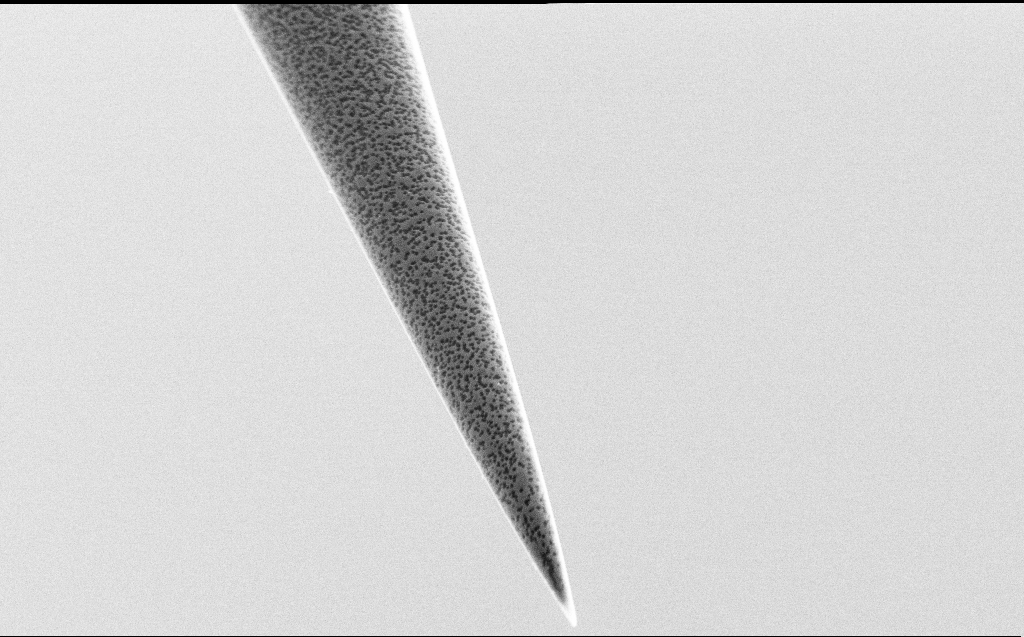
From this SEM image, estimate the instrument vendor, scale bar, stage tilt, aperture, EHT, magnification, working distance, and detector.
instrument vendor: Zeiss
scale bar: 2000 nm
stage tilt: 45°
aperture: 30 µm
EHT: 5 kV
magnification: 10 K X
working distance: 7 mm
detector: SE2